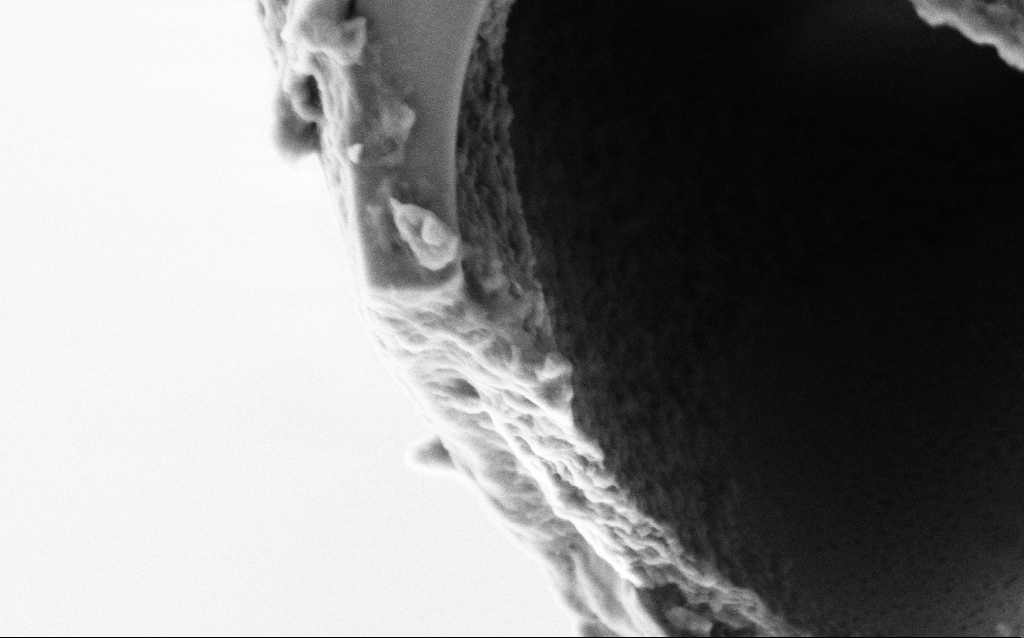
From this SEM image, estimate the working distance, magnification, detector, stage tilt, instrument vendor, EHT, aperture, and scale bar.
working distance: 6 mm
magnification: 75 K X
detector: SE2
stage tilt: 45°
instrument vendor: Zeiss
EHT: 2 kV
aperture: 30 µm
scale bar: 200 nm